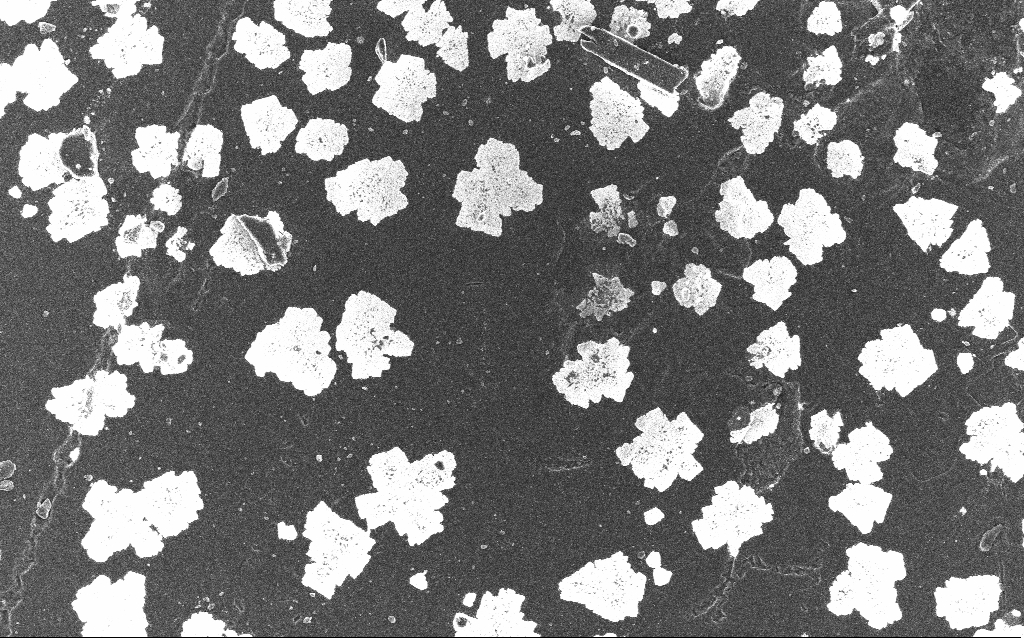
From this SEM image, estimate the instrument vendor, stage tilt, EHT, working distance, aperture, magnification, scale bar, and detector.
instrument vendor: Zeiss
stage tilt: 0°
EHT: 10 kV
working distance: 4.2 mm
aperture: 30 µm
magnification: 0.77 K X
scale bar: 20000 nm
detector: InLens